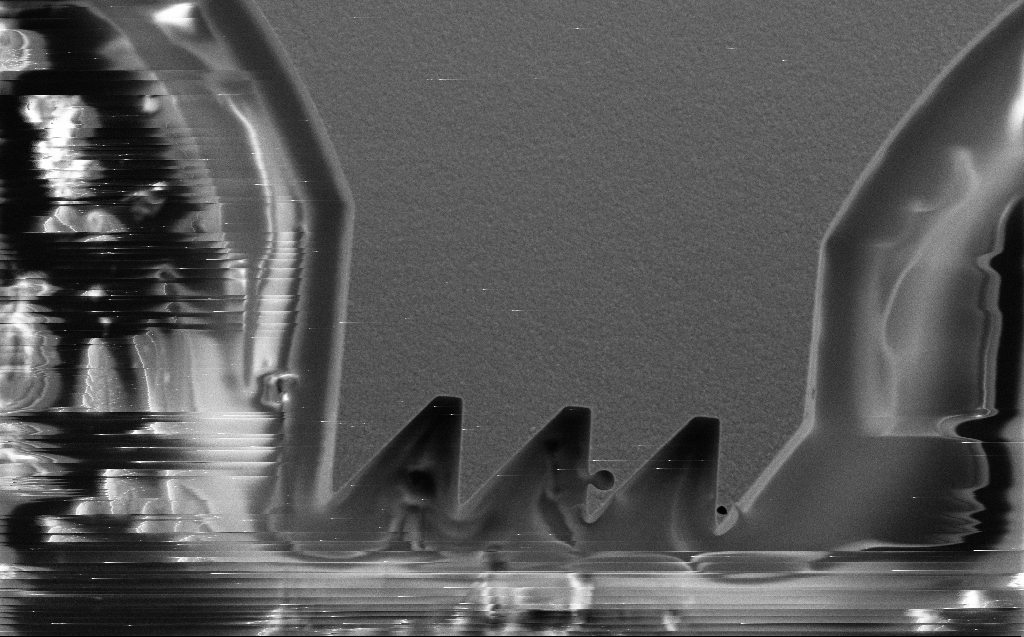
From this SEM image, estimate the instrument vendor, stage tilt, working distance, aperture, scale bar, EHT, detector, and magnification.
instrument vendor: Zeiss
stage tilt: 0°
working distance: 8 mm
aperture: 30 µm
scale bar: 10000 nm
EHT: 10 kV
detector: SE2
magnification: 4.19 K X